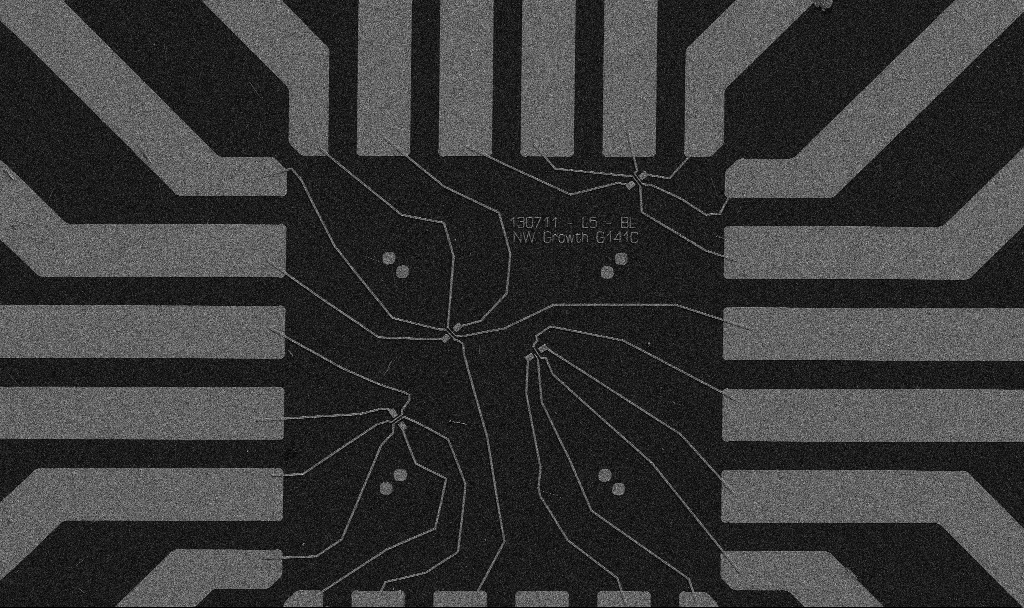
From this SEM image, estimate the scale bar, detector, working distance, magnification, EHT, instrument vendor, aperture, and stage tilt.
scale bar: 20000 nm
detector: SE2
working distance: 10.7 mm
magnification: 1 K X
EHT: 5 kV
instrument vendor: Zeiss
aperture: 30 µm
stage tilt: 0°